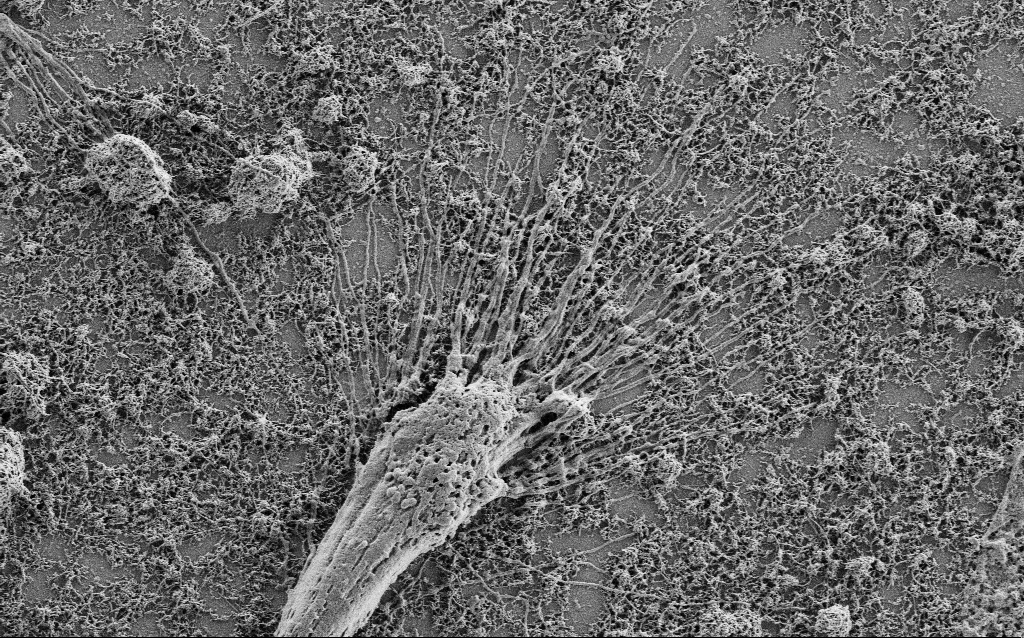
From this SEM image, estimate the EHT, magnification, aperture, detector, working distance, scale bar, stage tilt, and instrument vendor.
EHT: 1 kV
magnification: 10 K X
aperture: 30 µm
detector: SE2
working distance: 4 mm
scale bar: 2000 nm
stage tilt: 0°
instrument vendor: Zeiss